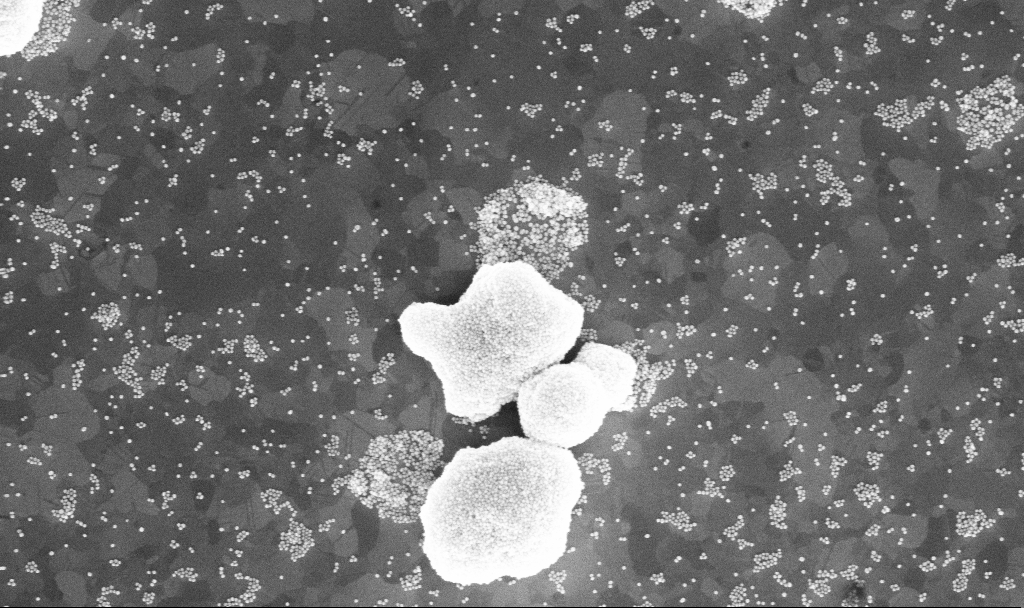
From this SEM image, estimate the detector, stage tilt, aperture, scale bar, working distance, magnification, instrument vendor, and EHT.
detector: InLens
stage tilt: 0°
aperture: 30 µm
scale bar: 200 nm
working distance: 3.9 mm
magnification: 80.58 K X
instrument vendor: Zeiss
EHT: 10 kV